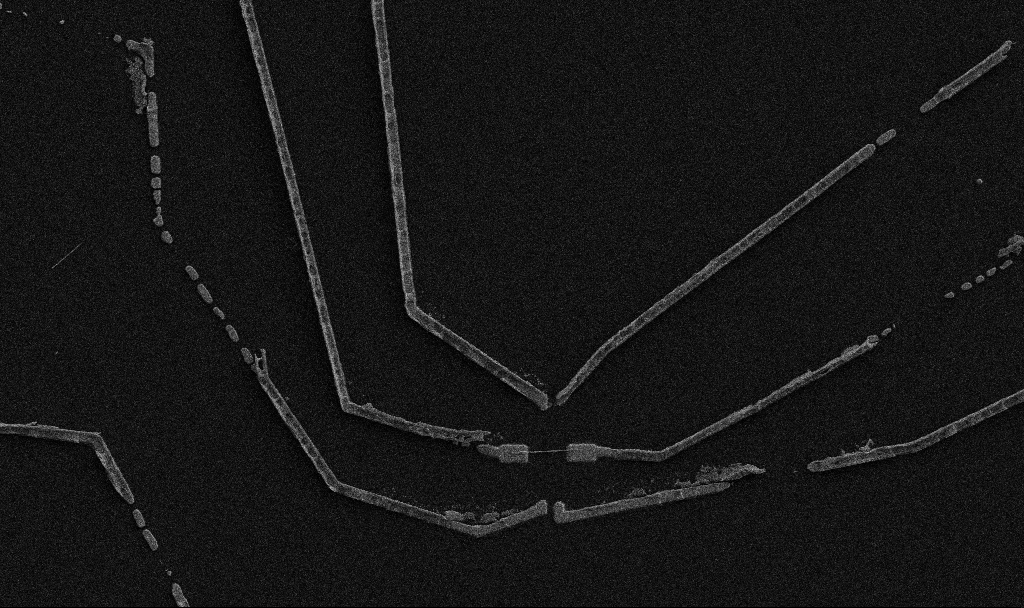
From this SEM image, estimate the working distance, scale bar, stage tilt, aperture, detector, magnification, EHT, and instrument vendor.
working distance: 10.7 mm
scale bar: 10000 nm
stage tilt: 0°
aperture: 30 µm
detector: SE2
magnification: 5 K X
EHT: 5 kV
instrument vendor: Zeiss